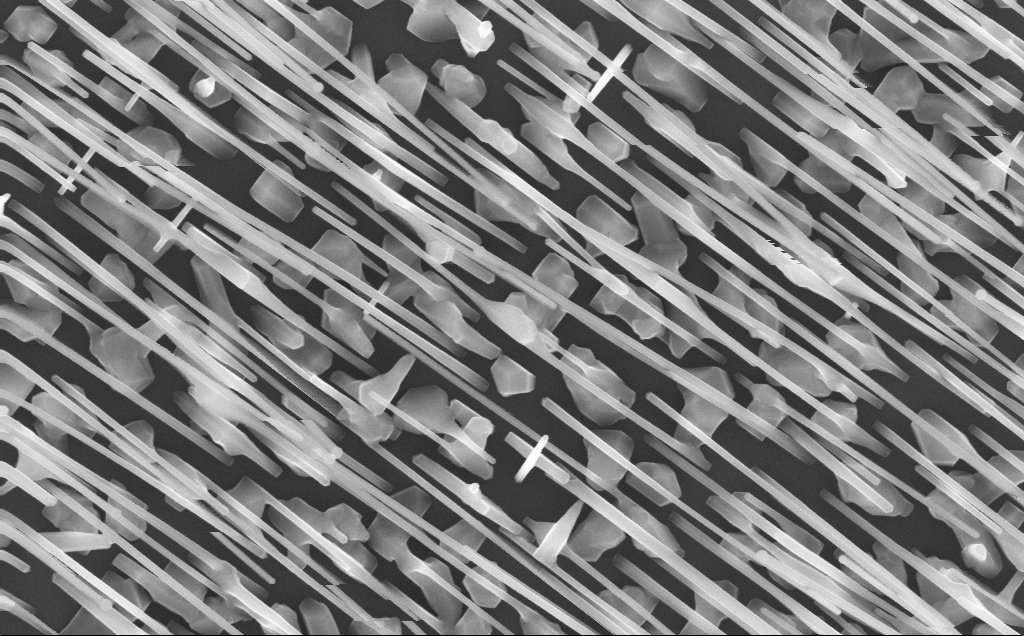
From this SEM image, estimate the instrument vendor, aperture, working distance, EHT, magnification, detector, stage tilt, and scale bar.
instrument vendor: Zeiss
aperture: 30 µm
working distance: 4 mm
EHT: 10 kV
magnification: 80 K X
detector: InLens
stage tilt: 0°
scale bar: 200 nm